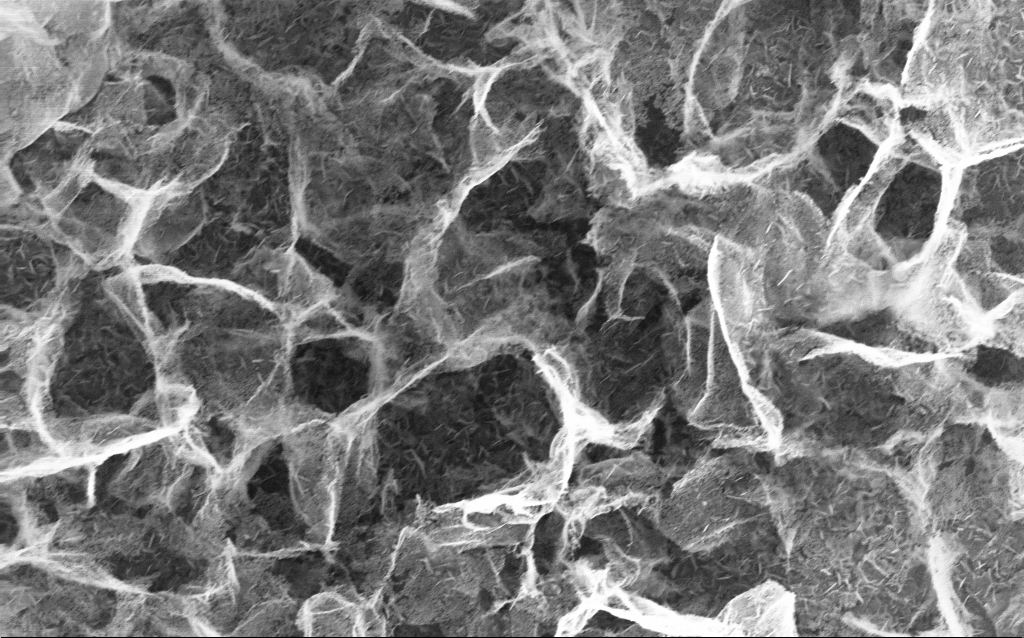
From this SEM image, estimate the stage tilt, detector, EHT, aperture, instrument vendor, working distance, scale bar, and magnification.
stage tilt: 0°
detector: InLens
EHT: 10 kV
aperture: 30 µm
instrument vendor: Zeiss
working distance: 2.8 mm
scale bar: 1000 nm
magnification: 40 K X